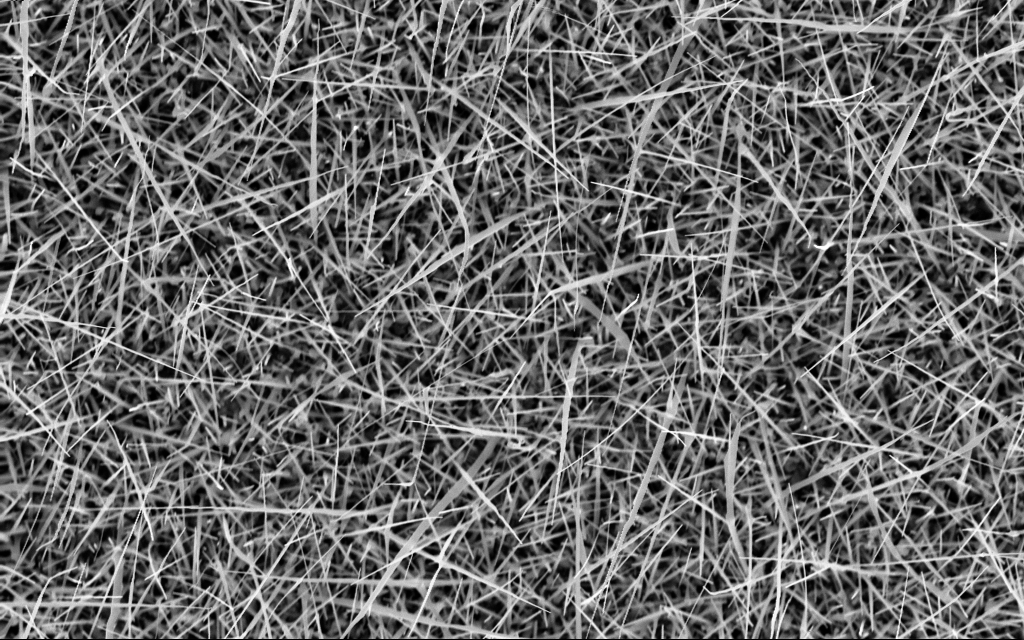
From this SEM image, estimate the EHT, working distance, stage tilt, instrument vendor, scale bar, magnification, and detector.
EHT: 10 kV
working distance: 5 mm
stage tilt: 0°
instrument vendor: Zeiss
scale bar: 2000 nm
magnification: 20 K X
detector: InLens